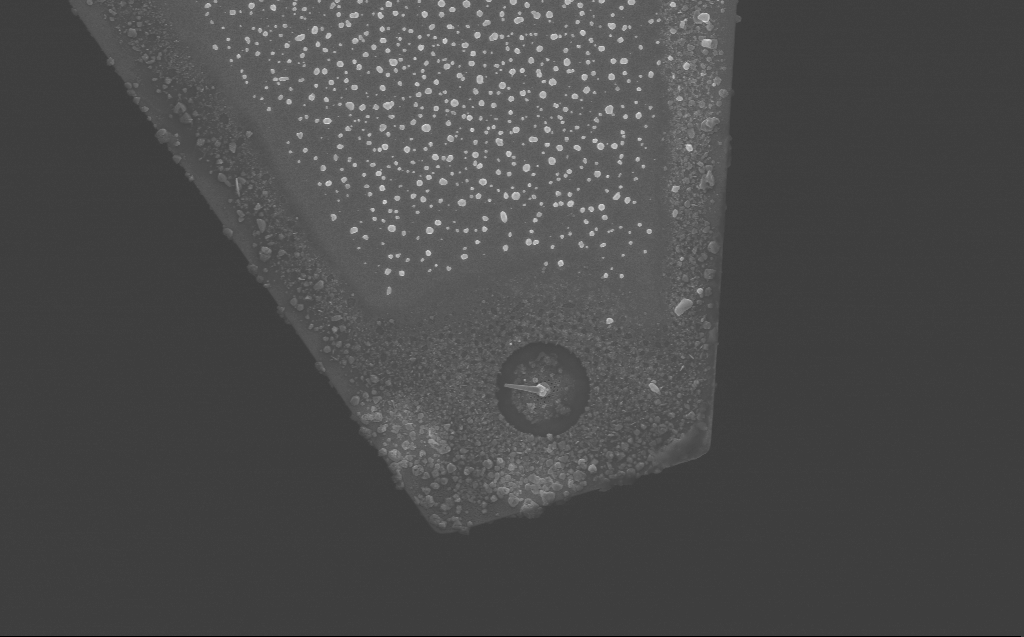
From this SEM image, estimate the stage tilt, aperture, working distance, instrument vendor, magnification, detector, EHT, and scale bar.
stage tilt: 0°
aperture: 30 µm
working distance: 6 mm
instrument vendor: Zeiss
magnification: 5.92 K X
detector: InLens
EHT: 10 kV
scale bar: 10000 nm